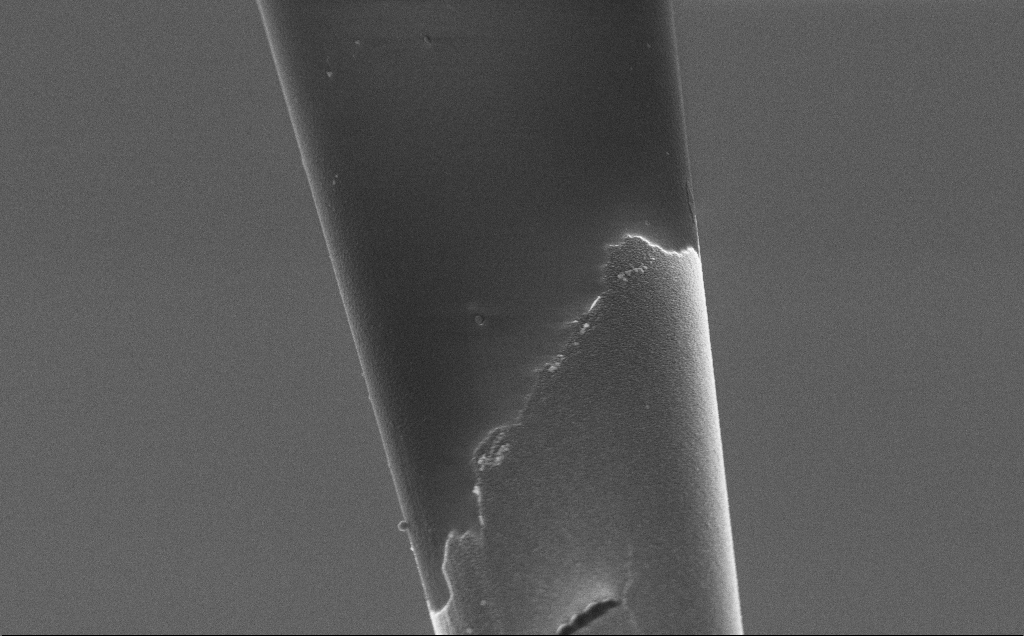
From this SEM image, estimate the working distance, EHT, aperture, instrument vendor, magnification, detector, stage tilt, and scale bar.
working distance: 7.8 mm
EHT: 3 kV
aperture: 30 µm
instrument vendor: Zeiss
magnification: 15 K X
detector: InLens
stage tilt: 45°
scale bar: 1000 nm